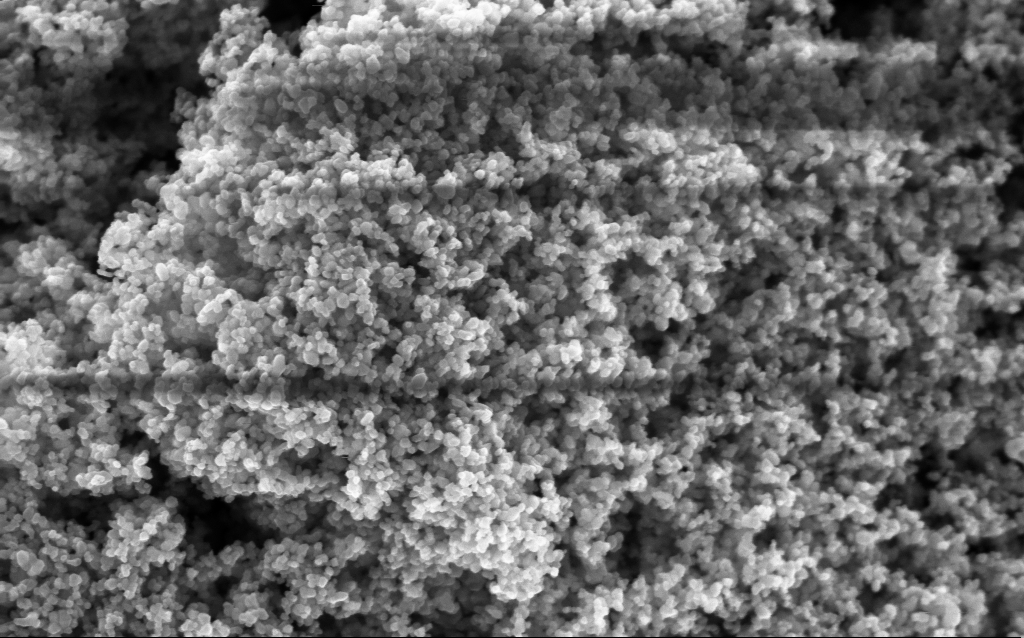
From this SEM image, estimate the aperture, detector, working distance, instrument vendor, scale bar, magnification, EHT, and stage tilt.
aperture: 30 µm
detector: InLens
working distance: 2.9 mm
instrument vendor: Zeiss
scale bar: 100 nm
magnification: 130 K X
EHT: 5 kV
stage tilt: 0°